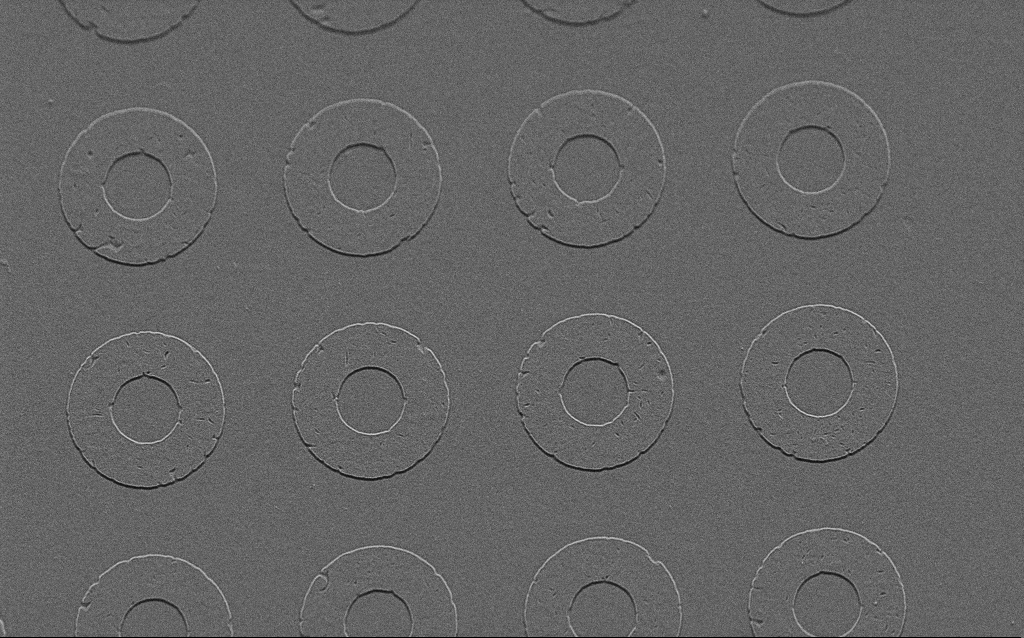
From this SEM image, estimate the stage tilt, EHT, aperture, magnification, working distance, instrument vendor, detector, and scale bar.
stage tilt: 45°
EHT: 1.5 kV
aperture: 30 µm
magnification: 8.28 K X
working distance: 6 mm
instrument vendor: Zeiss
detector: SE2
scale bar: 2000 nm